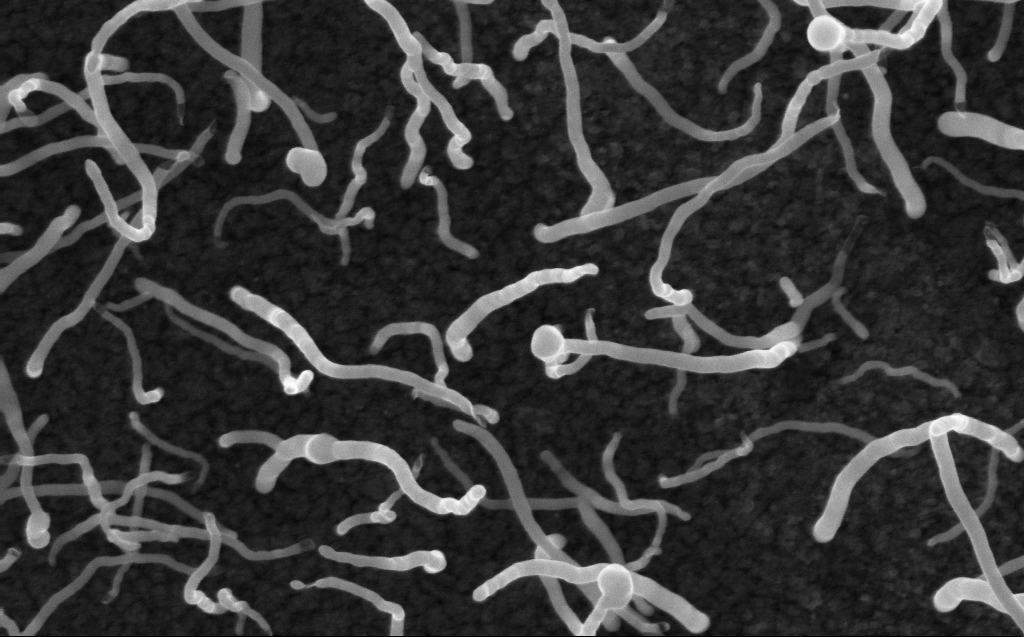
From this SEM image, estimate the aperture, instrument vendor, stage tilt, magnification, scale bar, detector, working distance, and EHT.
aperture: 30 µm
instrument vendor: Zeiss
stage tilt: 0°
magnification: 100 K X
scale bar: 200 nm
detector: InLens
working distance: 3 mm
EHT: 10 kV